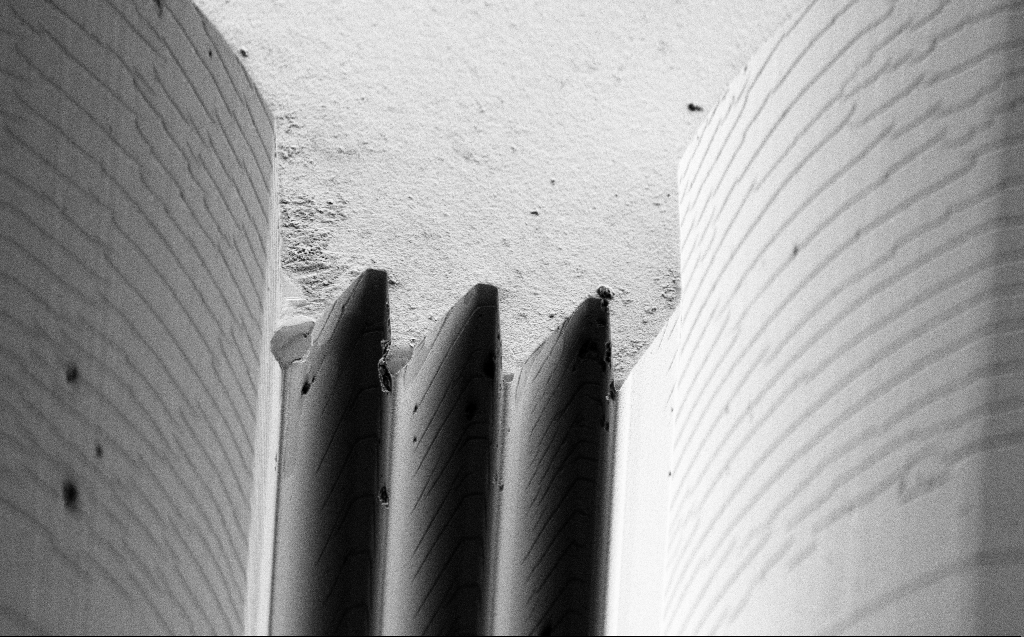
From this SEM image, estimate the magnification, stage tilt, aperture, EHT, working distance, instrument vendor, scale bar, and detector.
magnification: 3.61 K X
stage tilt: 45°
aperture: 30 µm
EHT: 3 kV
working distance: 8 mm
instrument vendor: Zeiss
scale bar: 10000 nm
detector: SE2